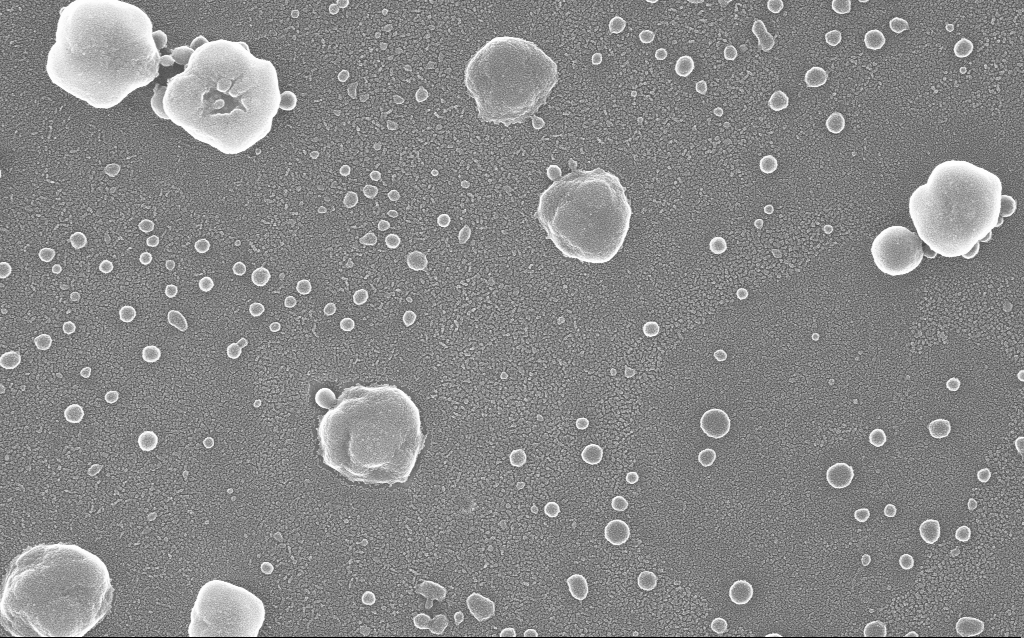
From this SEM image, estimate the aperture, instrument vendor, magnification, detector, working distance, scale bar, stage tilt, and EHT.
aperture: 30 µm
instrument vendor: Zeiss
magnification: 100 K X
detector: InLens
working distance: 1.5 mm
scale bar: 200 nm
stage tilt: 0°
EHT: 20 kV